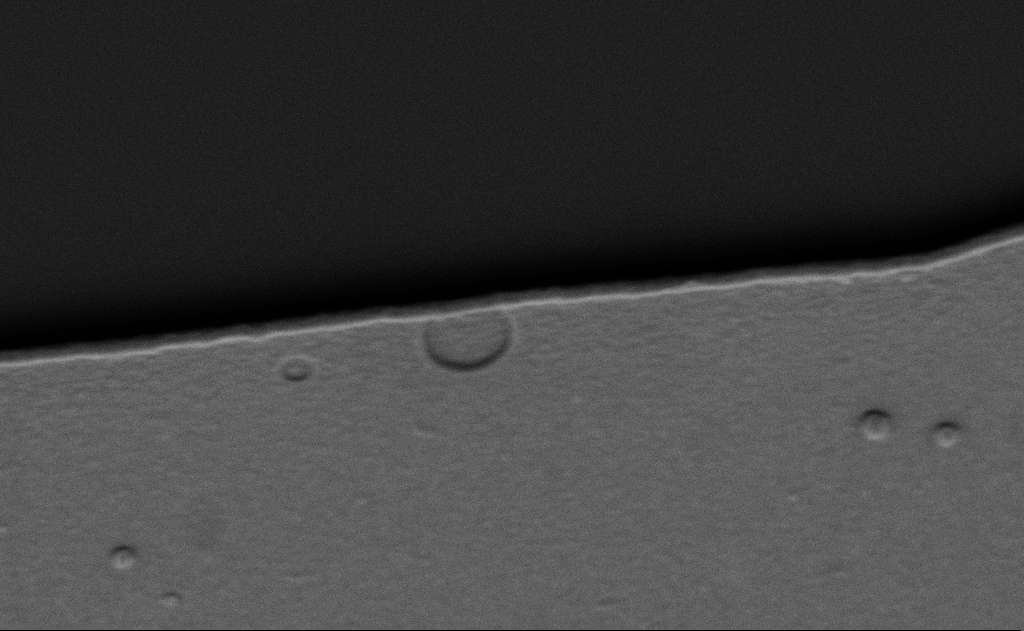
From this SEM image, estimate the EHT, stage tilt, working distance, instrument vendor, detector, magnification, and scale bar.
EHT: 5 kV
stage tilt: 39.8°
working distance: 8 mm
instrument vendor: Zeiss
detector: SE2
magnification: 44.91 K X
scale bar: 1000 nm